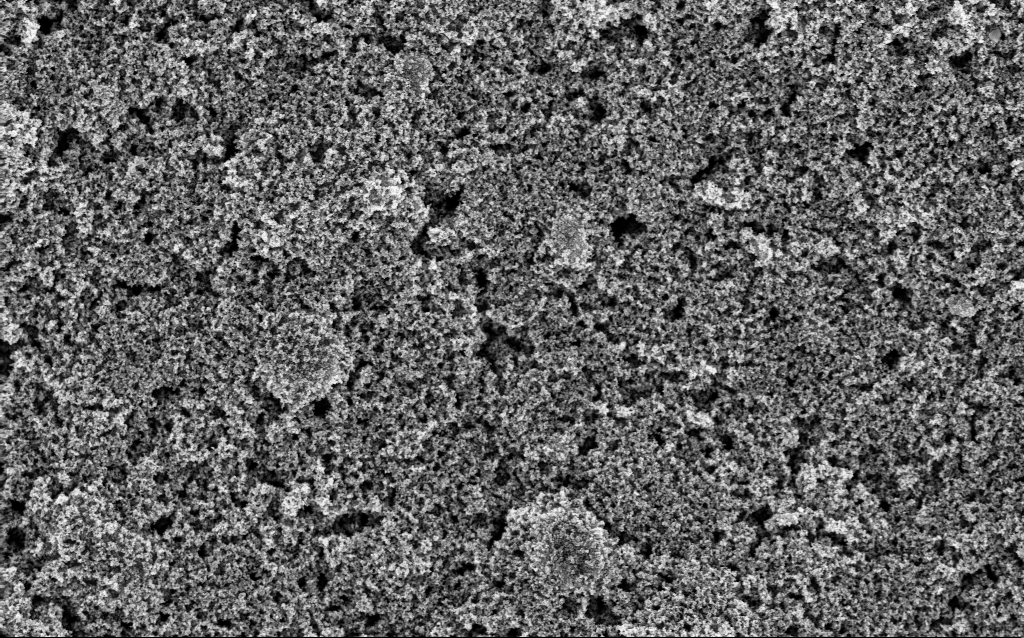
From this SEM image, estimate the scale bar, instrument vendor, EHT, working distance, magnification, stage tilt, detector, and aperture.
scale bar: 1000 nm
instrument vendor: Zeiss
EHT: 5 kV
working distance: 5.3 mm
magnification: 23.9 K X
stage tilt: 0°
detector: InLens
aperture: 30 µm